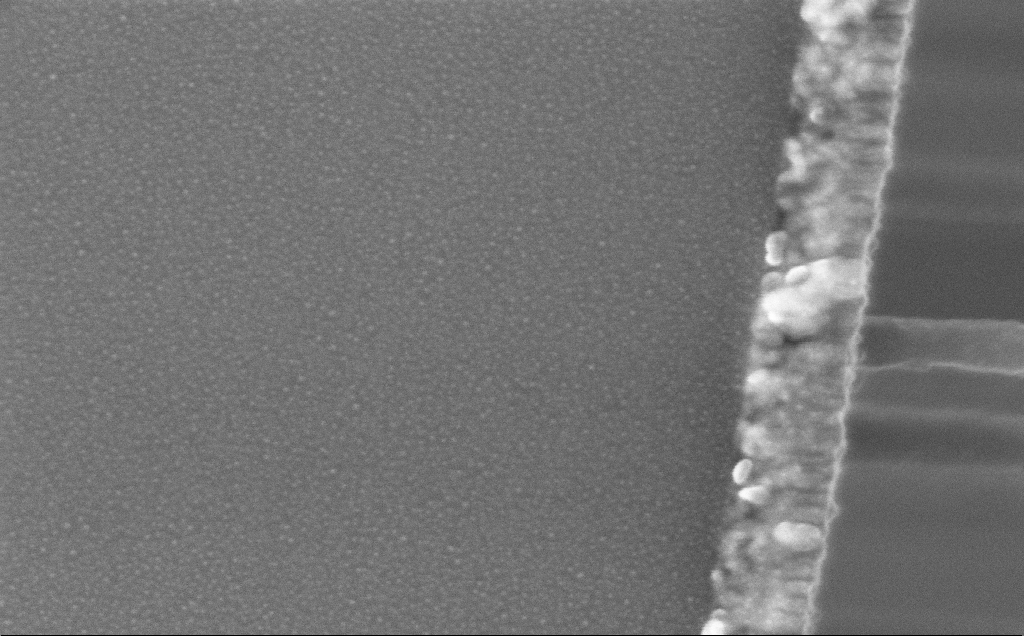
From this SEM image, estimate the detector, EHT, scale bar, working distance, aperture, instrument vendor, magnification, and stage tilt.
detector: InLens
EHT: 5 kV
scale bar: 200 nm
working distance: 12 mm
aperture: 30 µm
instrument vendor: Zeiss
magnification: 159.22 K X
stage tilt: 0°